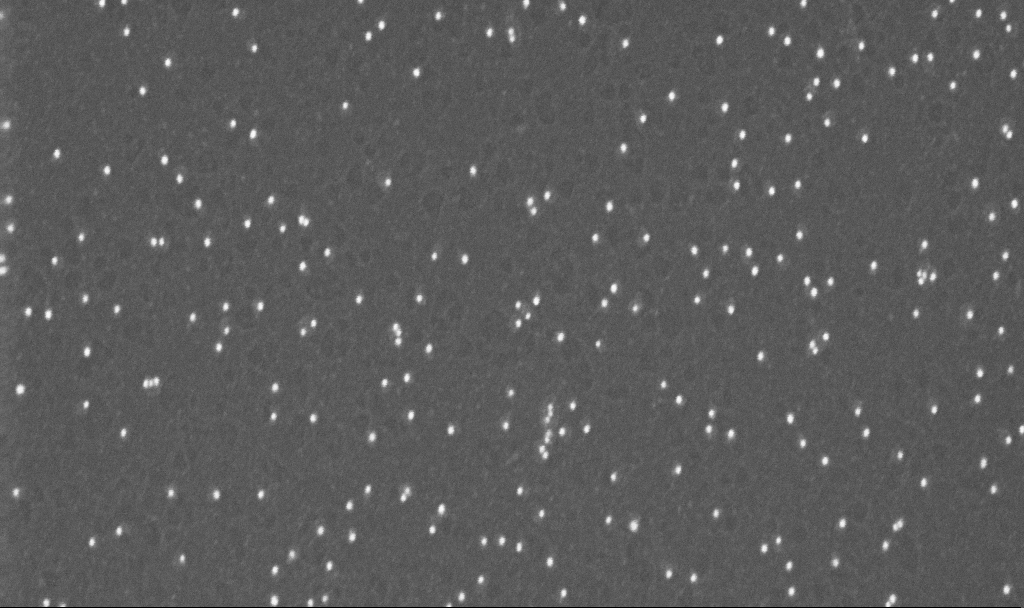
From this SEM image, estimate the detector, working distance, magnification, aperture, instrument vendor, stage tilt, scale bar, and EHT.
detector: InLens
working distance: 3.2 mm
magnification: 200 K X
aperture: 30 µm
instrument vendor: Zeiss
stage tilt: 0°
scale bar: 100 nm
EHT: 10 kV